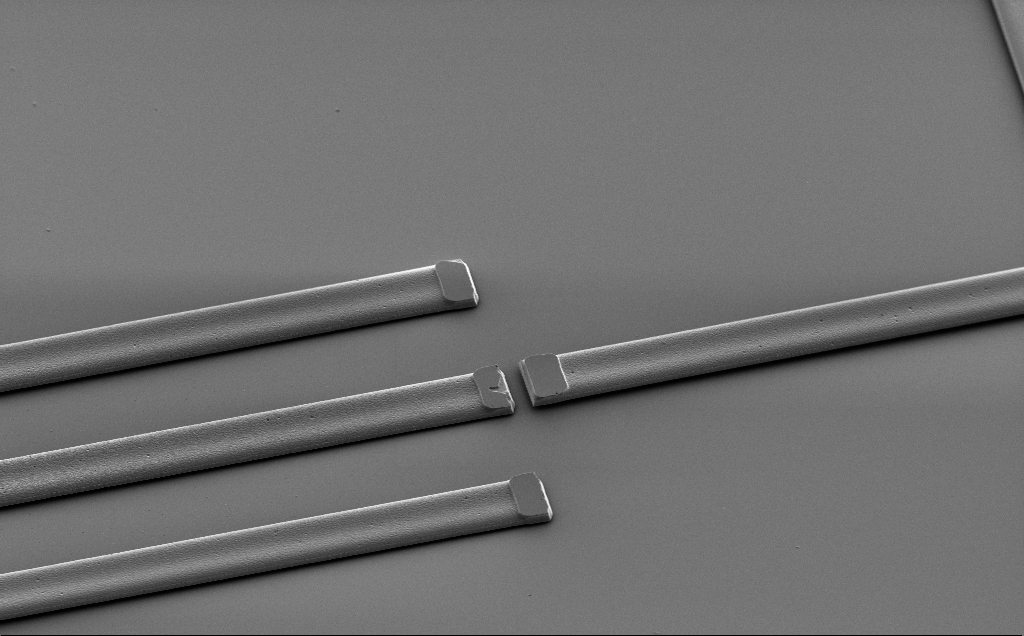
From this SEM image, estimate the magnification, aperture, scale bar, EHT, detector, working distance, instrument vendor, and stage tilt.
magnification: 1.4 K X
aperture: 30 µm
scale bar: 10000 nm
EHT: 2 kV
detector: SE2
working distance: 10 mm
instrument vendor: Zeiss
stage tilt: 43°